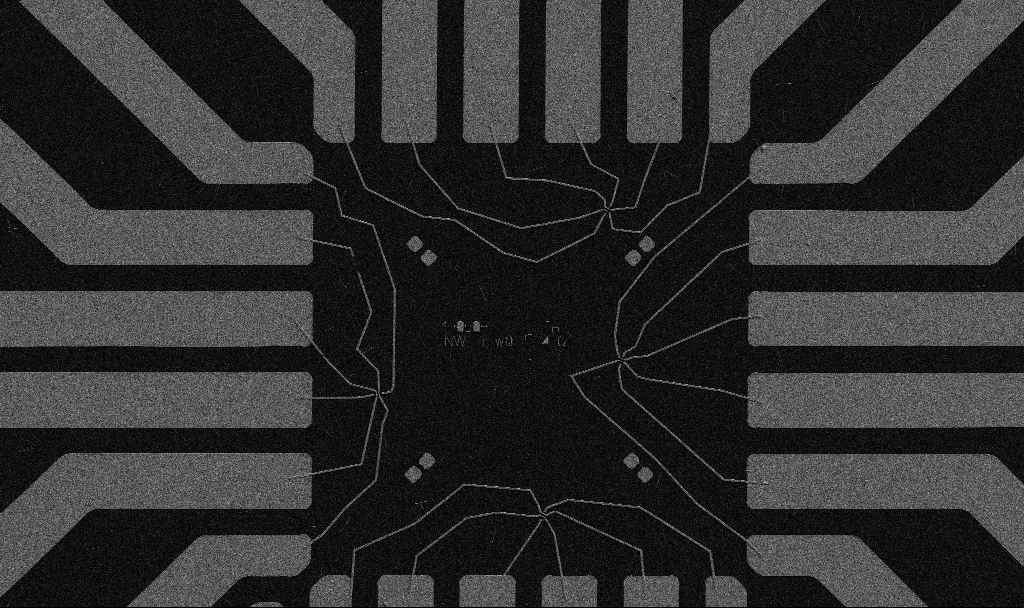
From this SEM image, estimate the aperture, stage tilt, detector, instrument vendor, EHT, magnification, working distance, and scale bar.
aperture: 30 µm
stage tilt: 0°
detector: SE2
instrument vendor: Zeiss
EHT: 5 kV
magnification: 1 K X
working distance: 10.7 mm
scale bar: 20000 nm